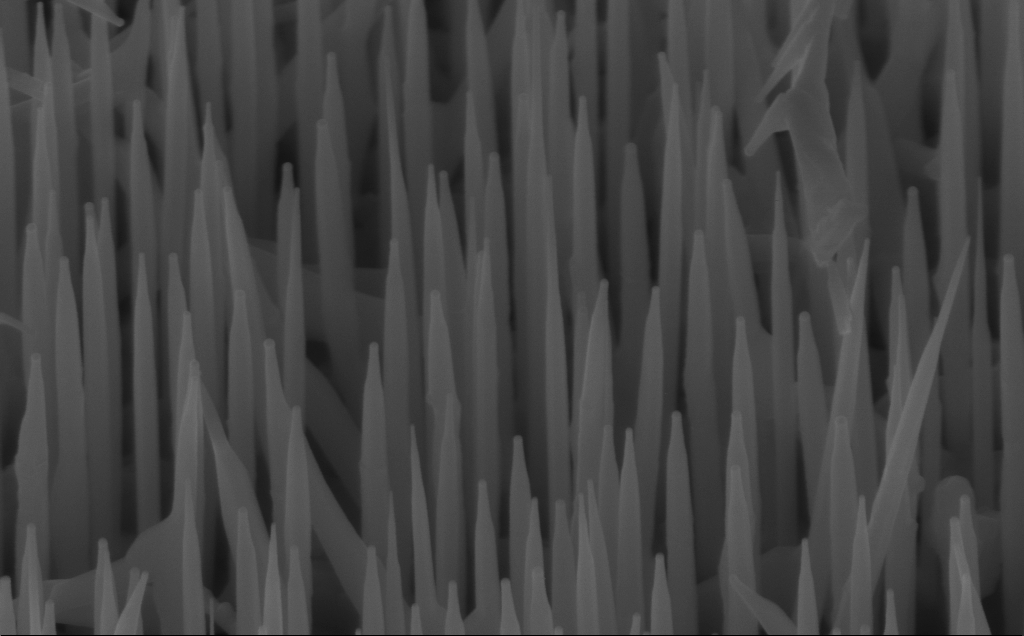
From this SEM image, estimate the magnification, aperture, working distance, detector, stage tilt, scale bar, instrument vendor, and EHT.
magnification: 80 K X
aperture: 30 µm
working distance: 7 mm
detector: InLens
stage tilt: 45°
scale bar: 200 nm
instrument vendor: Zeiss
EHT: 10 kV